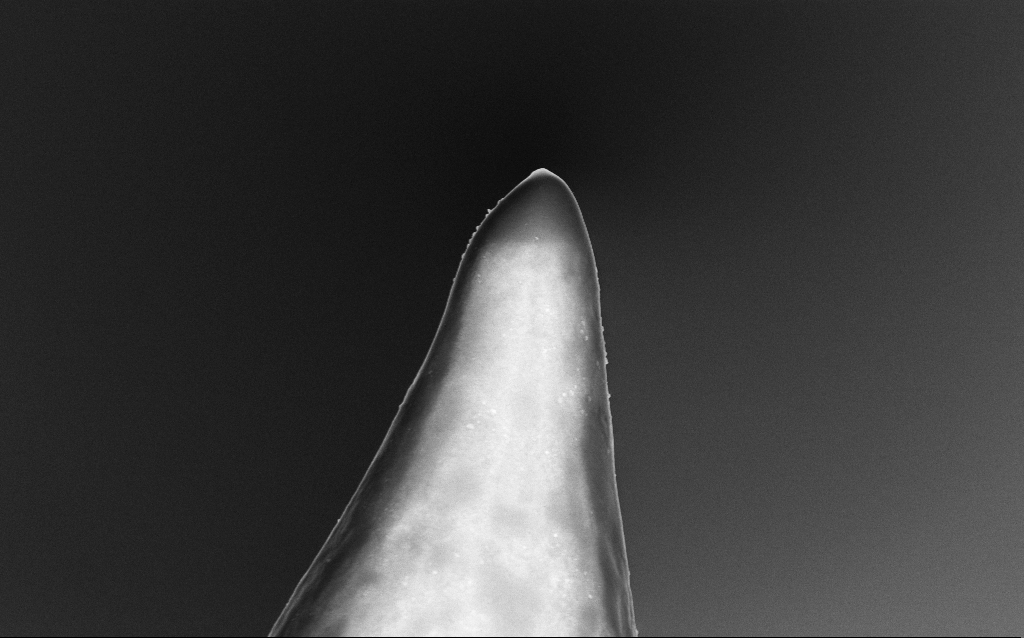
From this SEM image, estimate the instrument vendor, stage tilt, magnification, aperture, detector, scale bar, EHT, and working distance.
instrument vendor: Zeiss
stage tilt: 0°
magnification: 40.48 K X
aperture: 30 µm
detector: InLens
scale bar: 1000 nm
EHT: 5 kV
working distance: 3.1 mm